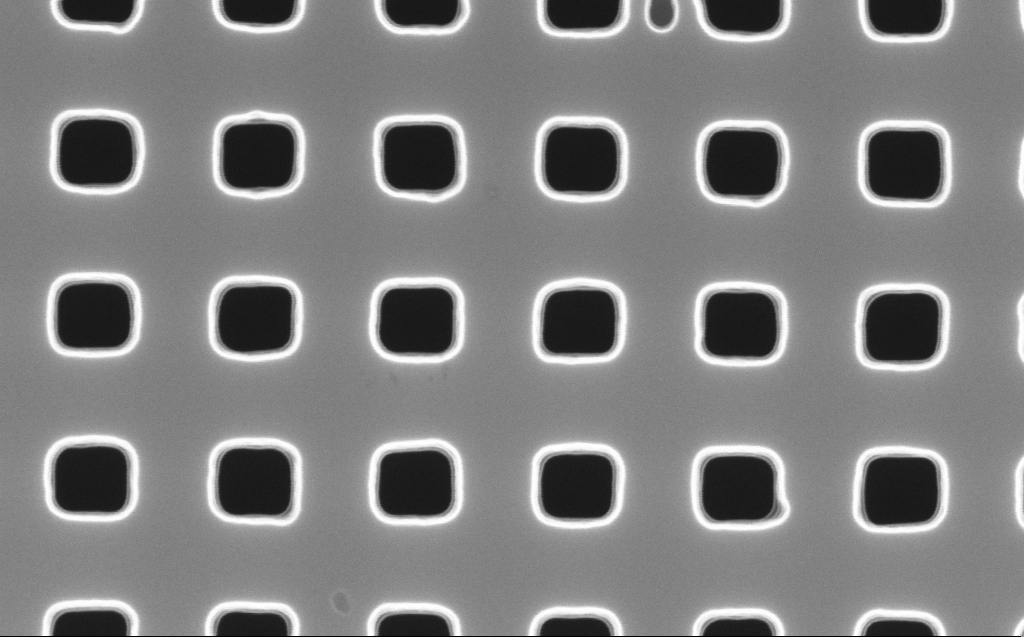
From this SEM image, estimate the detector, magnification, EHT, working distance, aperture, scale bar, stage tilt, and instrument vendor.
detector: InLens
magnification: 120 K X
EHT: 10 kV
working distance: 6 mm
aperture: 30 µm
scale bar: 100 nm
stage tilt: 0°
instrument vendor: Zeiss